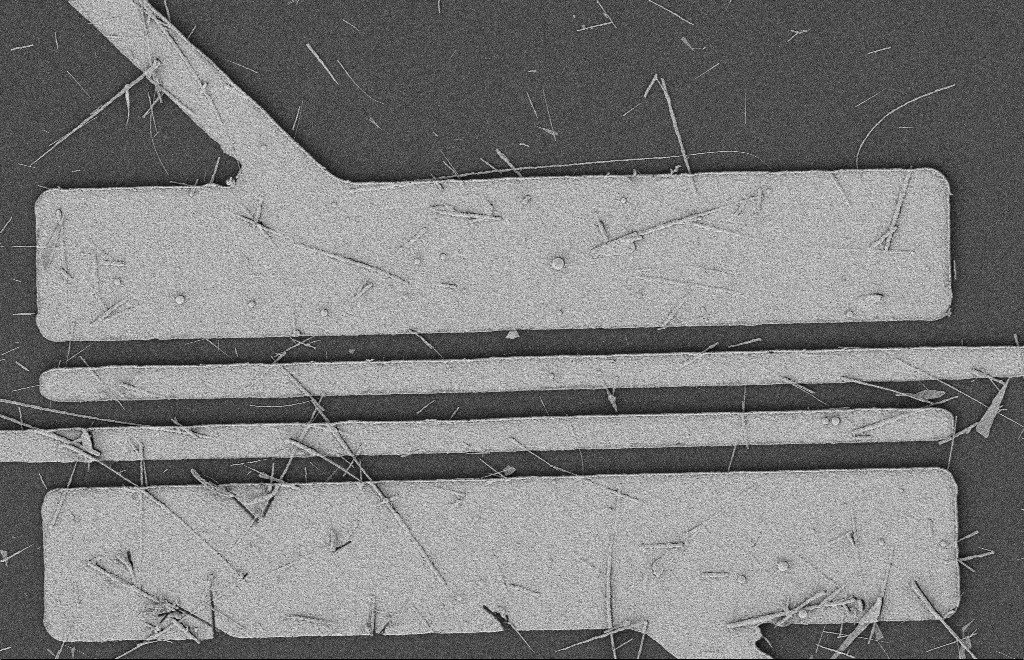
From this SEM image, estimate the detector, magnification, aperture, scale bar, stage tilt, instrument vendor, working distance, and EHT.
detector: SE2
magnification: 5.46 K X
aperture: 20 µm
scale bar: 2000 nm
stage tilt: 0°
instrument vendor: Zeiss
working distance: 12 mm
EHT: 2 kV